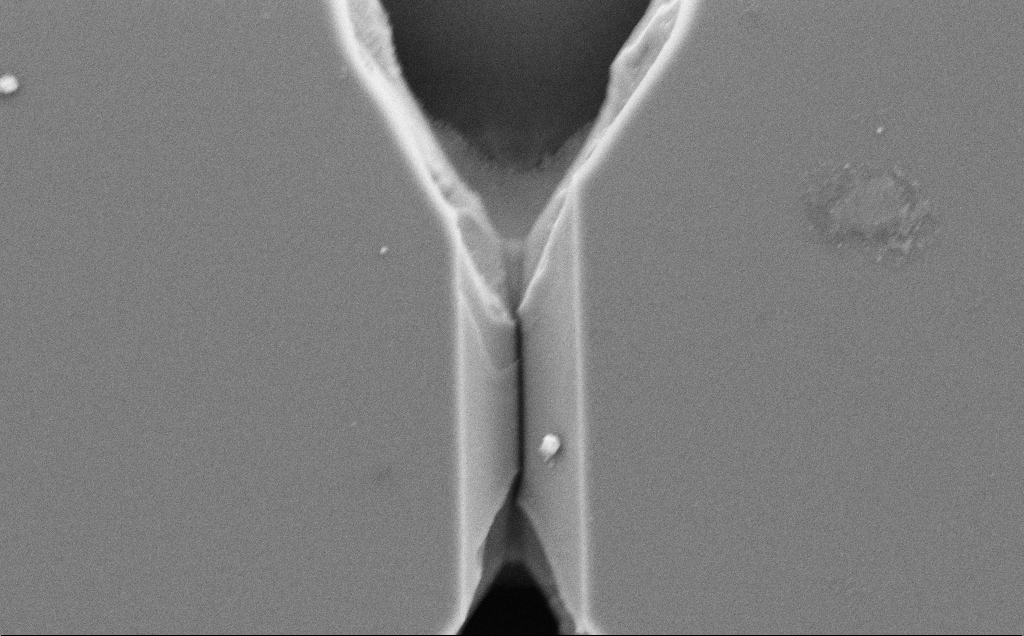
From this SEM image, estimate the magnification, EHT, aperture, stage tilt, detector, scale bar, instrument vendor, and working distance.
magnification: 19.43 K X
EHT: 5 kV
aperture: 30 µm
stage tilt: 0°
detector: SE2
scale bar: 2000 nm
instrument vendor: Zeiss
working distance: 10 mm